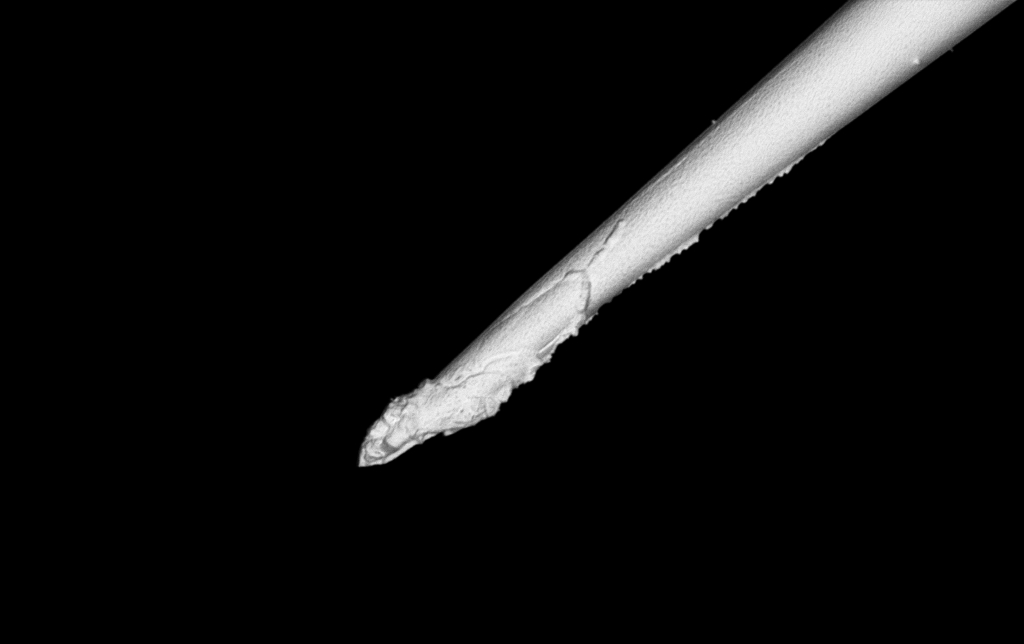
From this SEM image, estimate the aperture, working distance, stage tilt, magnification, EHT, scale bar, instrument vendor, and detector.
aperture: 30 µm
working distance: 7.5 mm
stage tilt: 0°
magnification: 5 K X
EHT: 3 kV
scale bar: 10000 nm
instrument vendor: Zeiss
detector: InLens